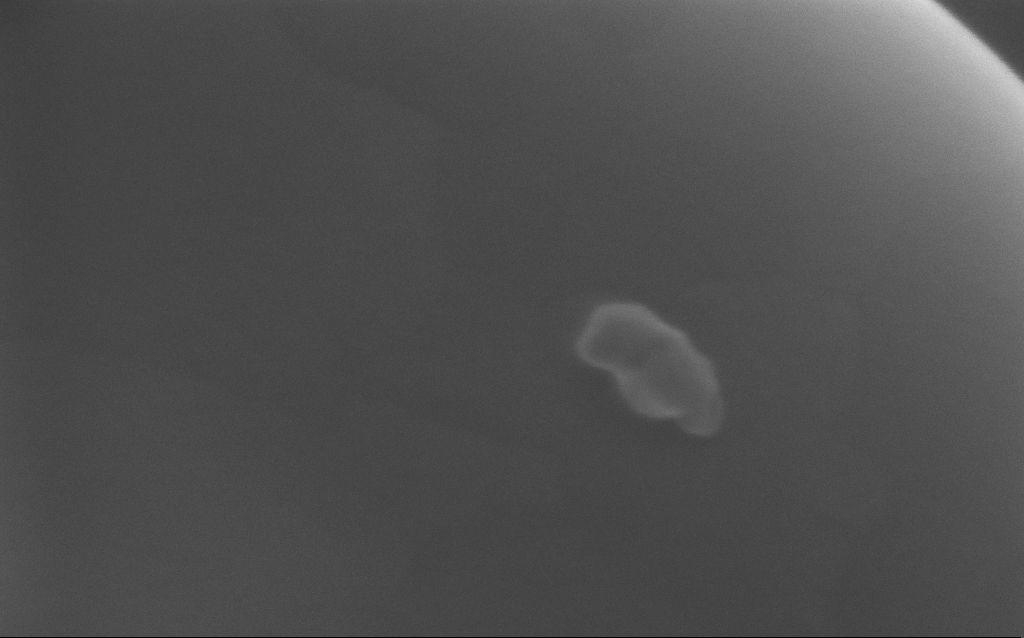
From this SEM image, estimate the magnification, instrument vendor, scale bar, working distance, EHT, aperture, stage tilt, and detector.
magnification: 597.11 K X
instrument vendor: Zeiss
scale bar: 100 nm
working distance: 3 mm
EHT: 5 kV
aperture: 30 µm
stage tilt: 0°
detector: InLens